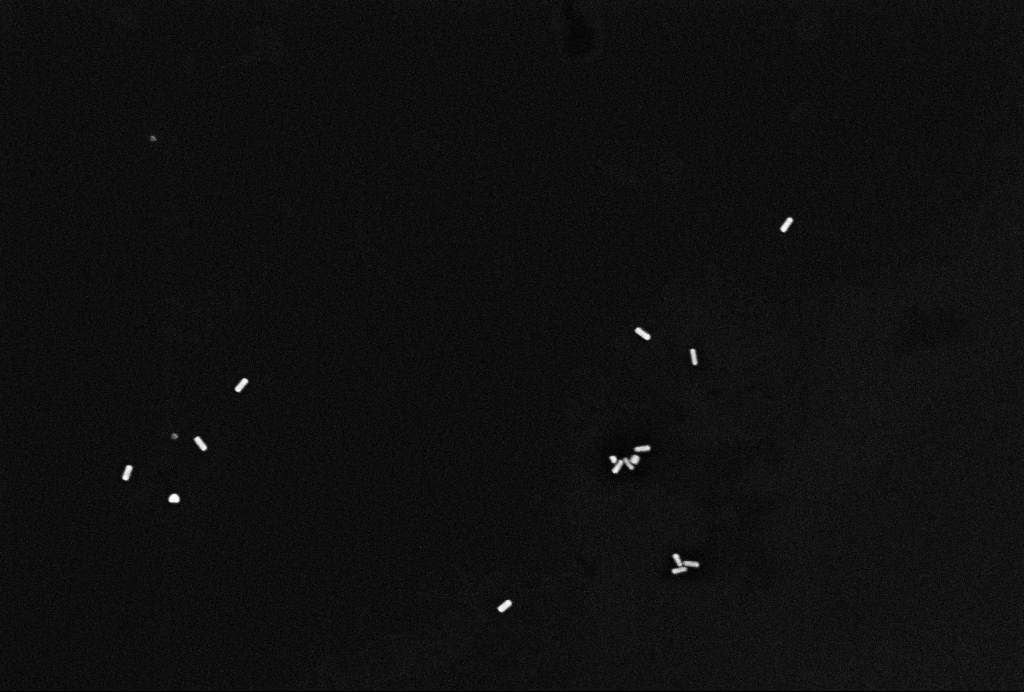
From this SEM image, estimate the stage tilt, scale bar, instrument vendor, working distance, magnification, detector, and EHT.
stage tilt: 0°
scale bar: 200 nm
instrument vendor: Zeiss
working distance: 3.2 mm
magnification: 80 K X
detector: InLens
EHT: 10 kV